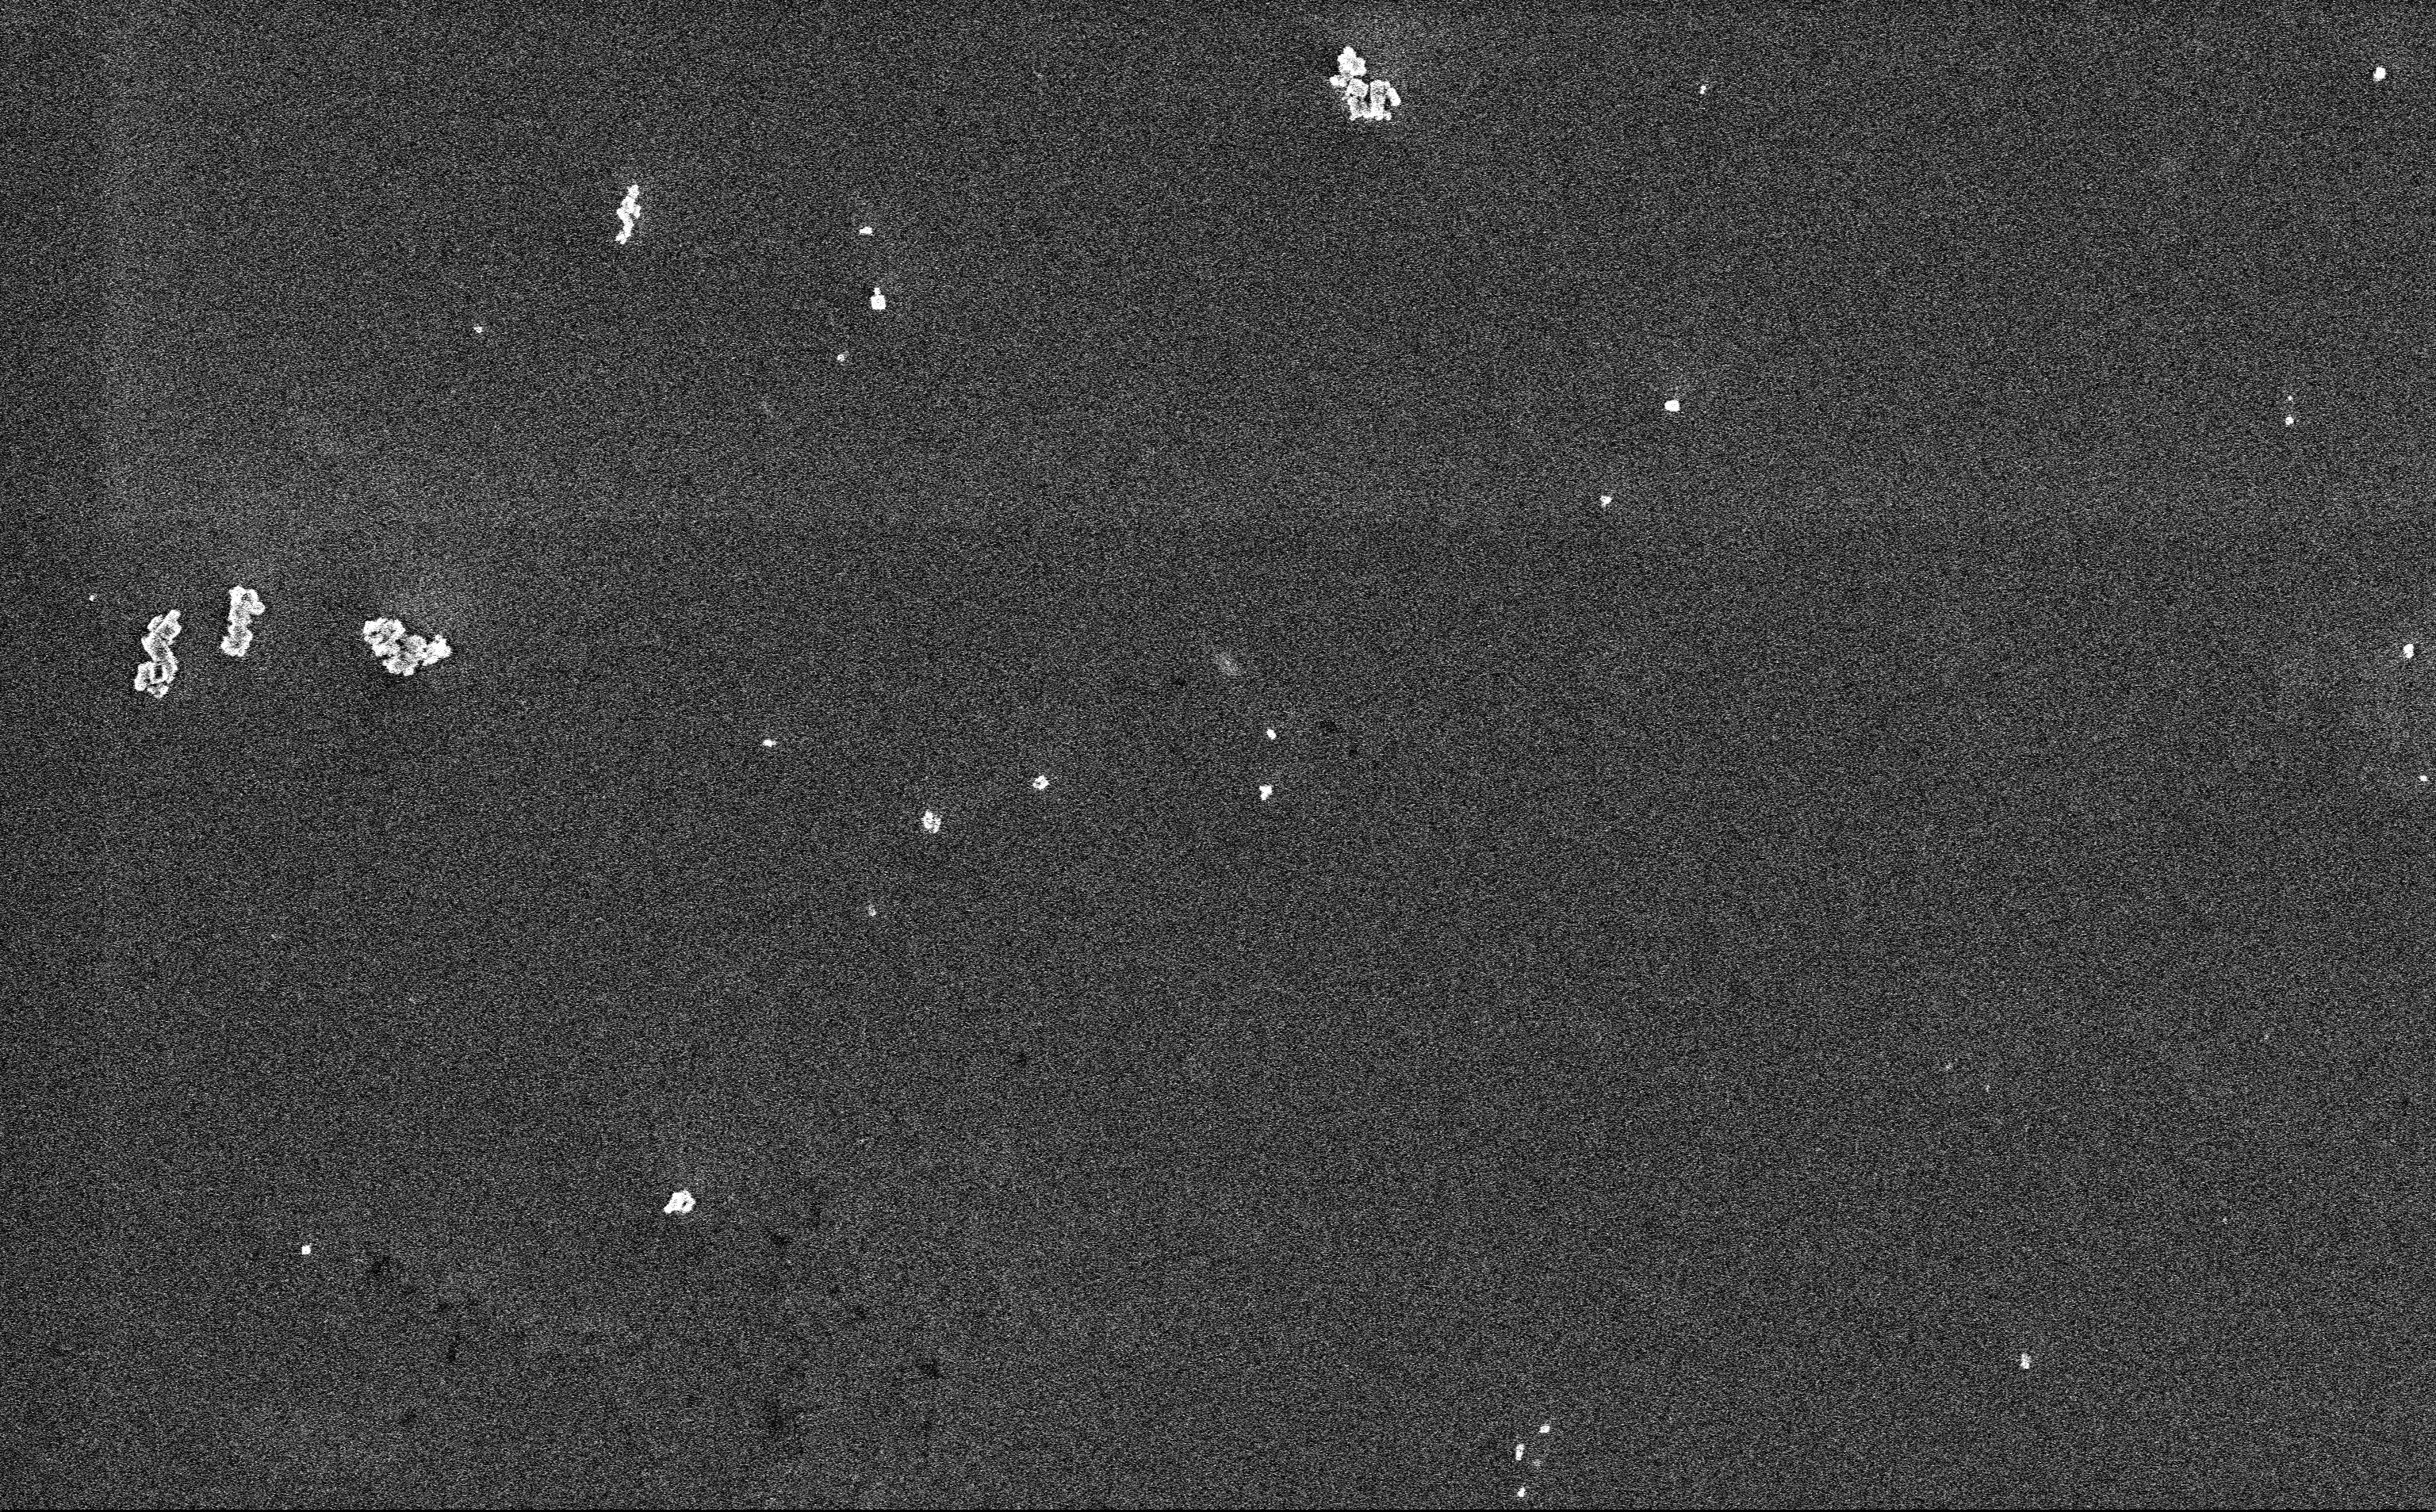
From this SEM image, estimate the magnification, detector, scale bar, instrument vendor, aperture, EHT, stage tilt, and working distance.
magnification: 12.85 K X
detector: InLens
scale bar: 2000 nm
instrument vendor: Zeiss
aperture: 30 µm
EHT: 3 kV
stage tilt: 0°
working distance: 3 mm